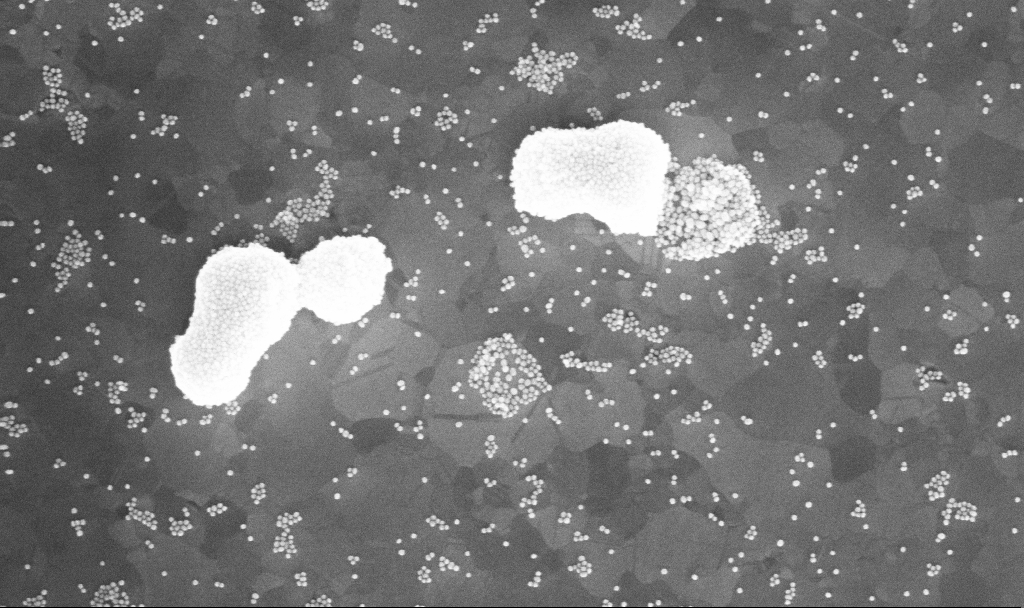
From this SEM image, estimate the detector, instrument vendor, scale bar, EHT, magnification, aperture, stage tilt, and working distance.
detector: InLens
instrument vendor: Zeiss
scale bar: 200 nm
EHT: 10 kV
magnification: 115.58 K X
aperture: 30 µm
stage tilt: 0°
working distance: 3.9 mm